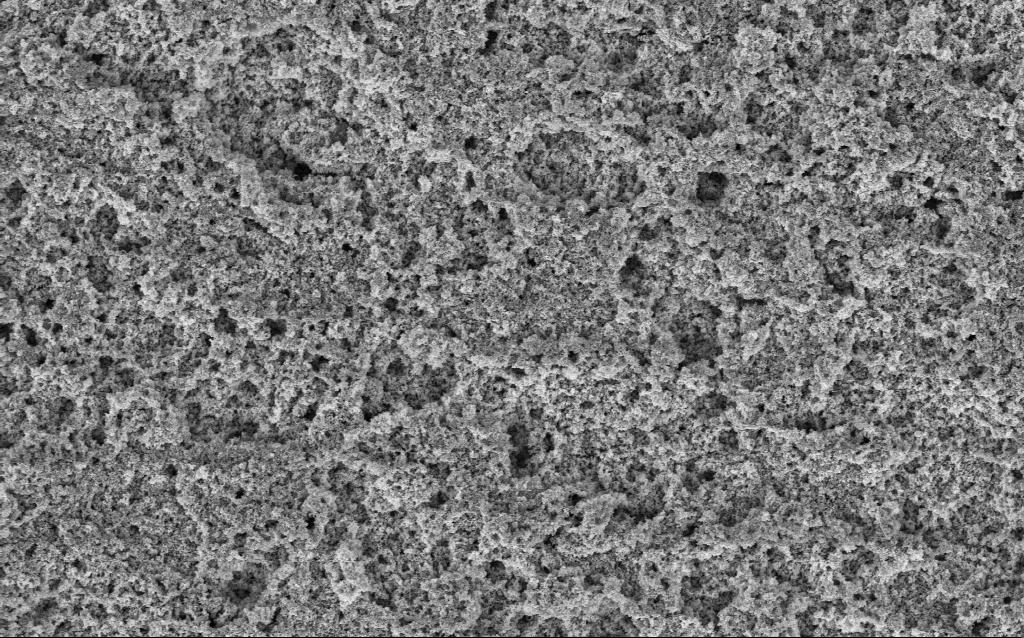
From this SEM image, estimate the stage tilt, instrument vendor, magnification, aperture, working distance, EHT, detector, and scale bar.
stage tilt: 0°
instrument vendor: Zeiss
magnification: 20.87 K X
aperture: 30 µm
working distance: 10 mm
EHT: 3 kV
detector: InLens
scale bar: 1000 nm